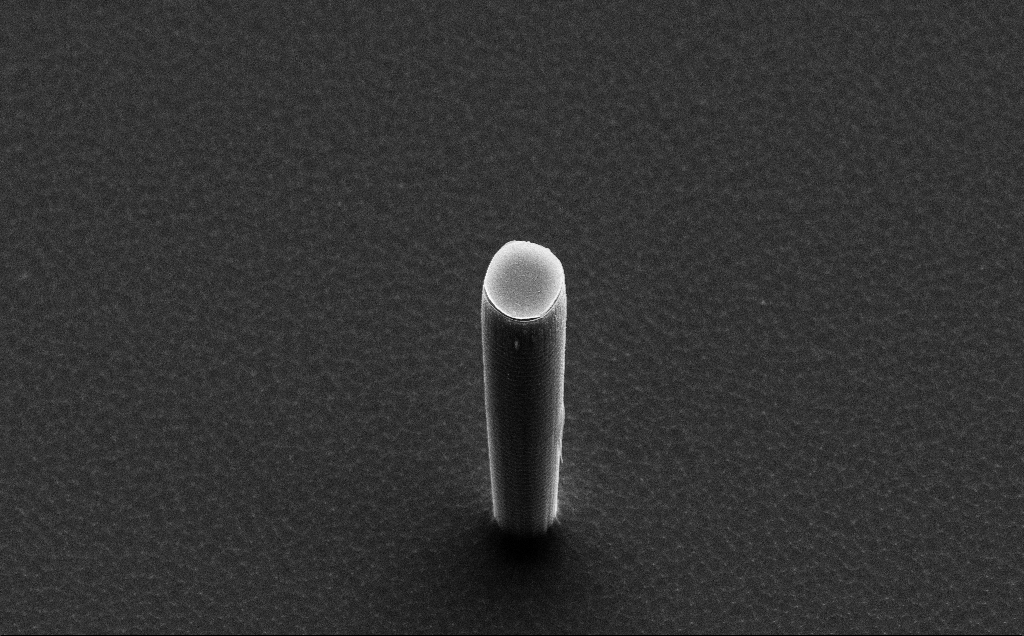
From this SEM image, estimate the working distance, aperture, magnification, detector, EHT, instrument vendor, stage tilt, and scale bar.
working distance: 10 mm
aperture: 30 µm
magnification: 4.5 K X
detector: SE2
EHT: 15 kV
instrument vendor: Zeiss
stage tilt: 50°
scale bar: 10000 nm